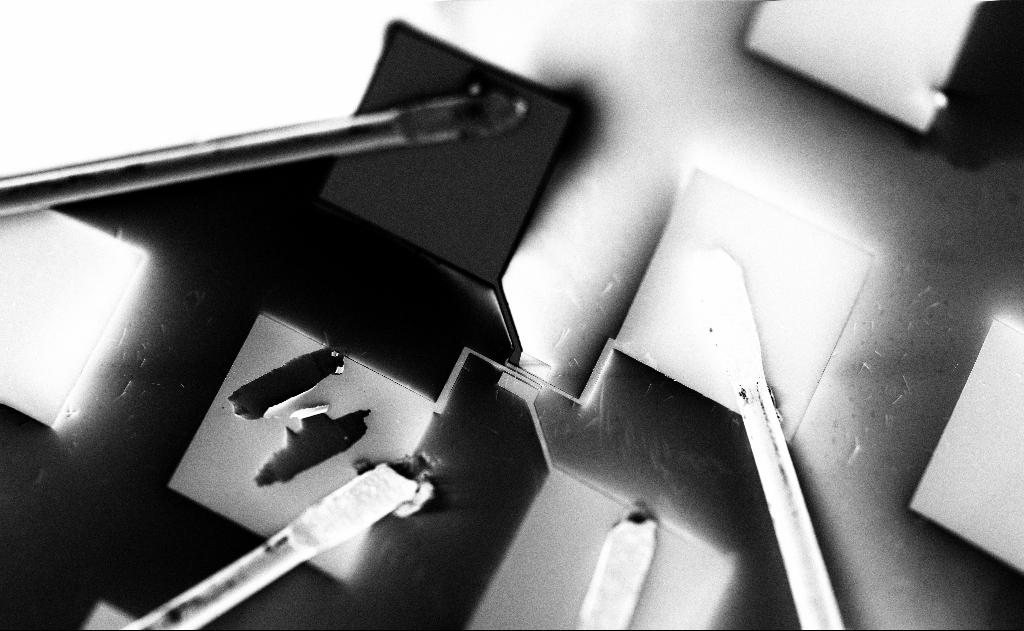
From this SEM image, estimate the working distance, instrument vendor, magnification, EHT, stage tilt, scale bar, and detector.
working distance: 20 mm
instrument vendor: Zeiss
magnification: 0.482 K X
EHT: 5 kV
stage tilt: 0°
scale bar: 100000 nm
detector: SE2